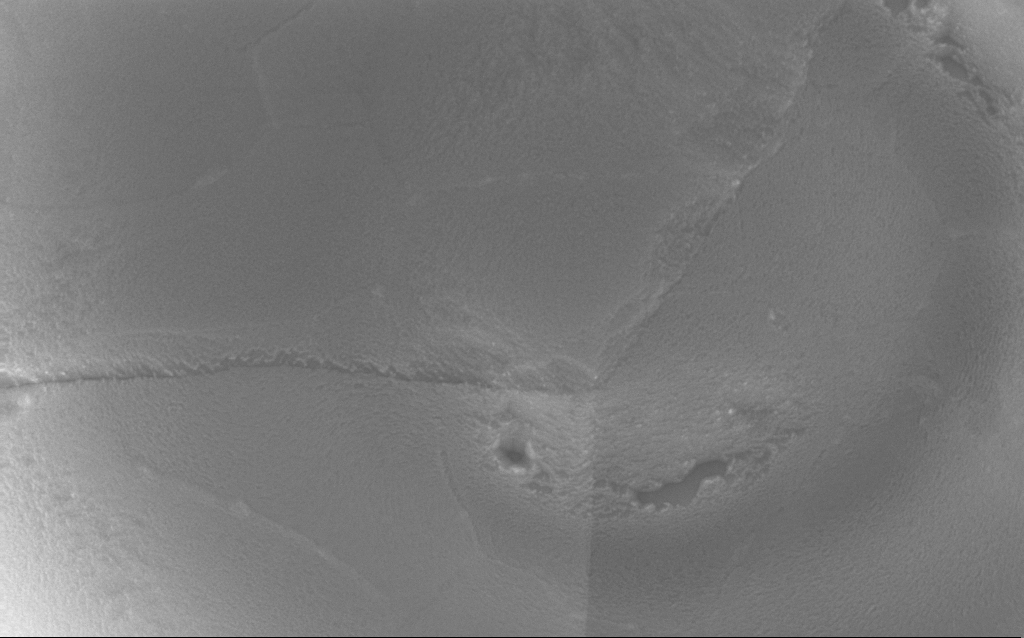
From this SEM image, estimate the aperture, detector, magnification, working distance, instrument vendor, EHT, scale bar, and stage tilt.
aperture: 30 µm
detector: InLens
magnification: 177.19 K X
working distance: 2 mm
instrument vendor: Zeiss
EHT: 10 kV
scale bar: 200 nm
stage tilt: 0°